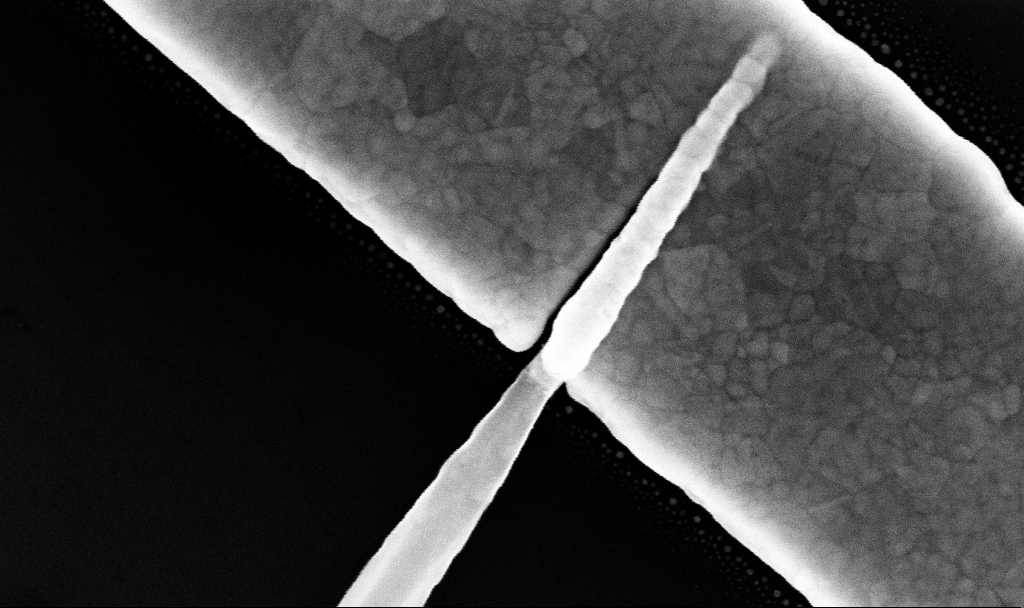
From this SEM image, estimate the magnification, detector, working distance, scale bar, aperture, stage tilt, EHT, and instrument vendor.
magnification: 184.97 K X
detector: InLens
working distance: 7.7 mm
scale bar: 200 nm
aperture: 30 µm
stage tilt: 0°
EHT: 10 kV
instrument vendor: Zeiss